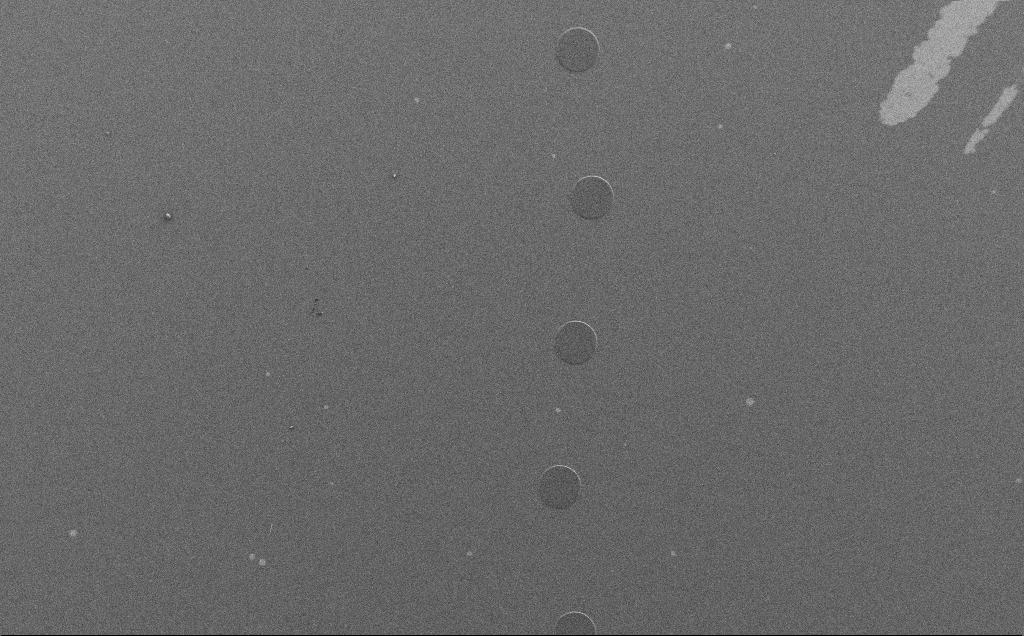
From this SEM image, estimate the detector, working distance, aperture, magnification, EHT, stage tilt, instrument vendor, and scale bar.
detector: SE2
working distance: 4 mm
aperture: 30 µm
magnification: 0.264 K X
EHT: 1.5 kV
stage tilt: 0°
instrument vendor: Zeiss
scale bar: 100000 nm